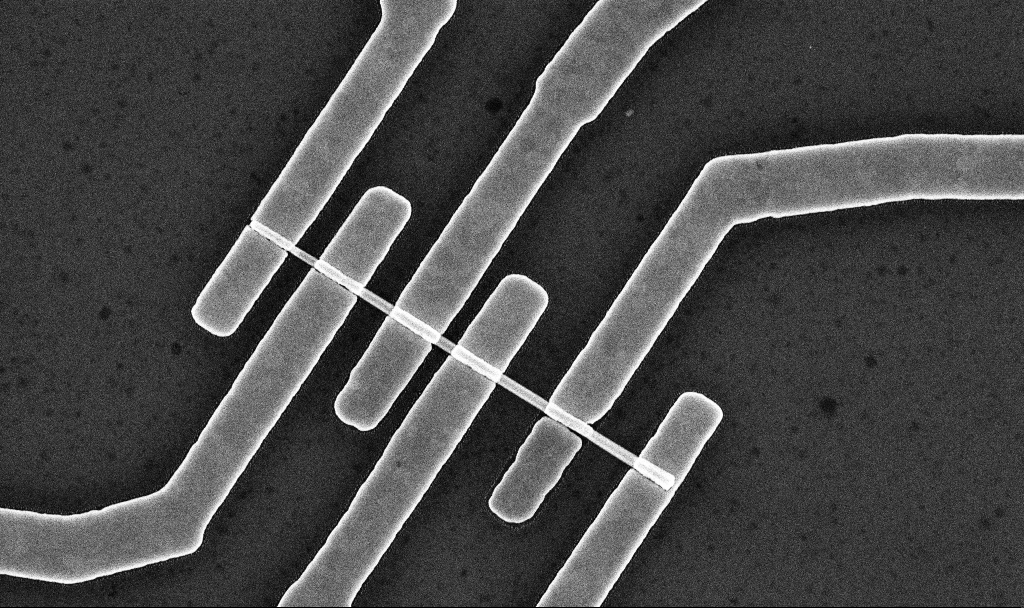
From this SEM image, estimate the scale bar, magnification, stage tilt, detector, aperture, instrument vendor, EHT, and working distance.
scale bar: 2000 nm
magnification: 29 K X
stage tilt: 0°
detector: InLens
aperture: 30 µm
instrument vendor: Zeiss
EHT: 10 kV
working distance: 7.6 mm